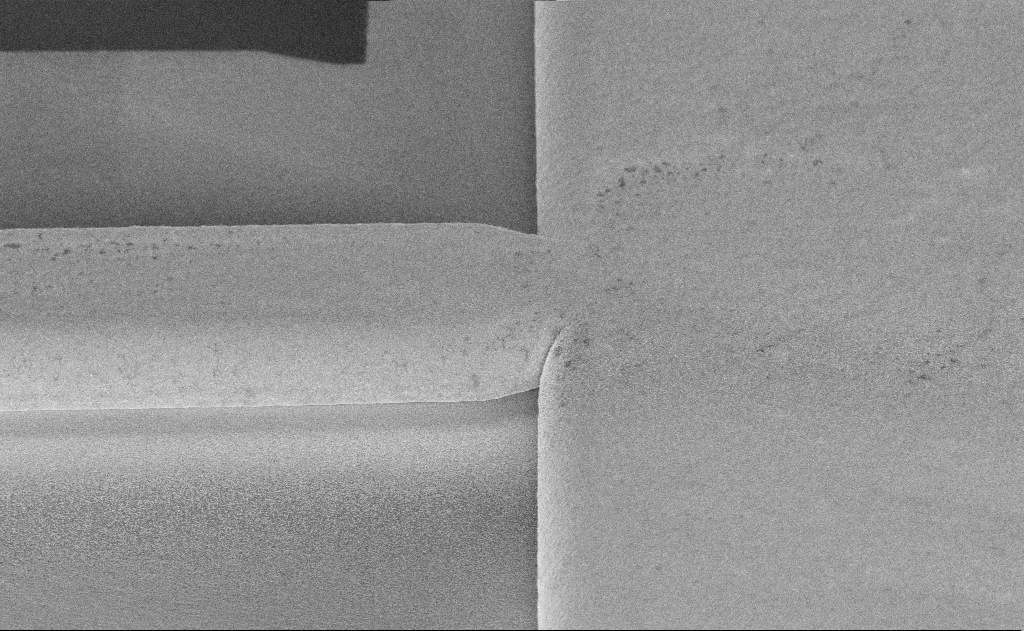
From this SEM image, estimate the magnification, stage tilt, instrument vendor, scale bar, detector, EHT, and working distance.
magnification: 5.42 K X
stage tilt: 60°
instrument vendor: Zeiss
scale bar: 10000 nm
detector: InLens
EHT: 10 kV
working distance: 14 mm